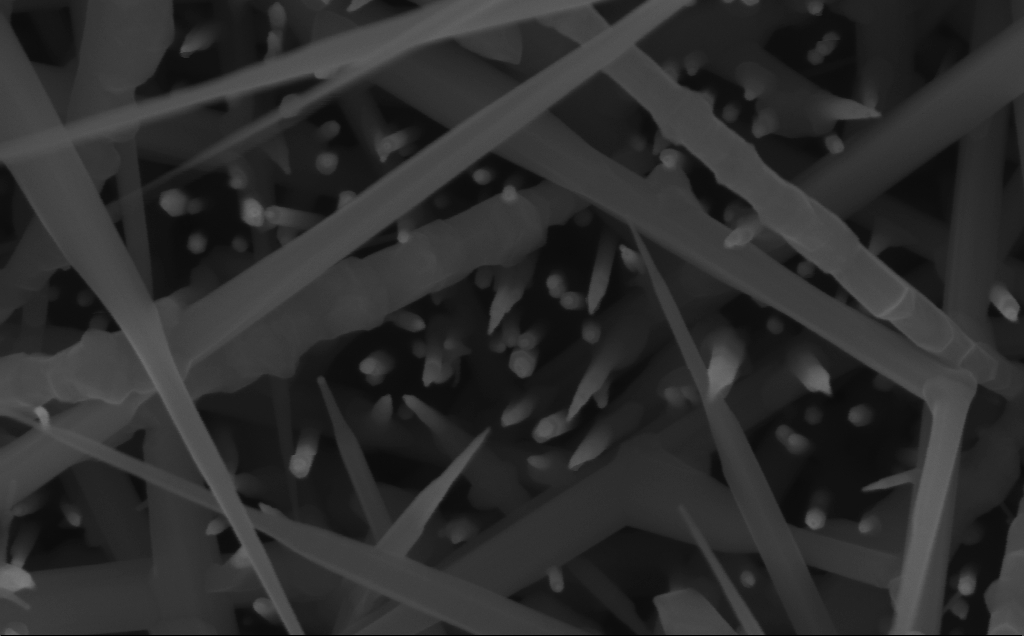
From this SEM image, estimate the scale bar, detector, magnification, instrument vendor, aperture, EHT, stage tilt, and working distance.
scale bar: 100 nm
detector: InLens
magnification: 188.3 K X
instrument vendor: Zeiss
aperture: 30 µm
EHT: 10 kV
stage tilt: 0°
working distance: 6 mm